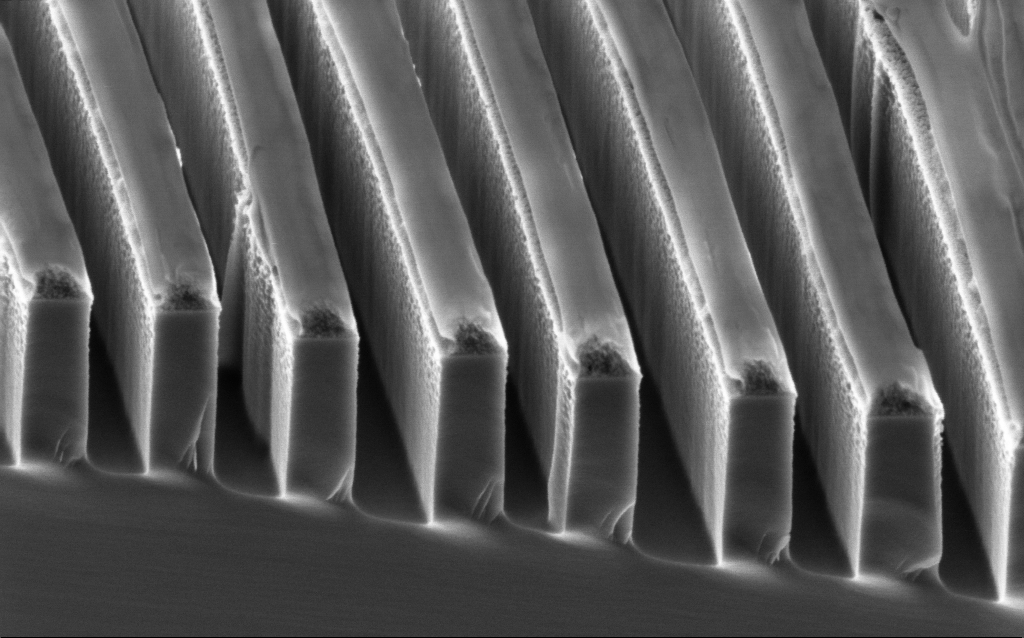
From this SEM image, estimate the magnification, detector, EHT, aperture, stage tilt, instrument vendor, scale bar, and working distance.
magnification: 94.43 K X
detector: InLens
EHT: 2 kV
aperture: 30 µm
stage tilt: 45°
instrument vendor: Zeiss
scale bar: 200 nm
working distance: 3.6 mm